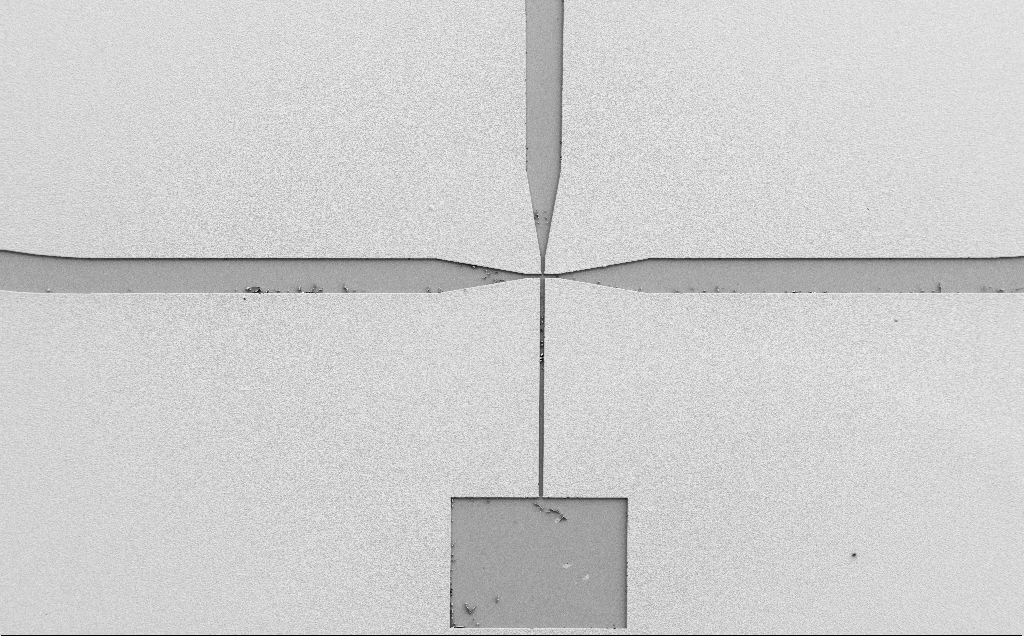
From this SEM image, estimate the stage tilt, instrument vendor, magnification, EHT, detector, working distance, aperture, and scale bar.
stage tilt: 0°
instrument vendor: Zeiss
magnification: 0.161 K X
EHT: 5 kV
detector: SE2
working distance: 13 mm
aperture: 30 µm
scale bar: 100000 nm